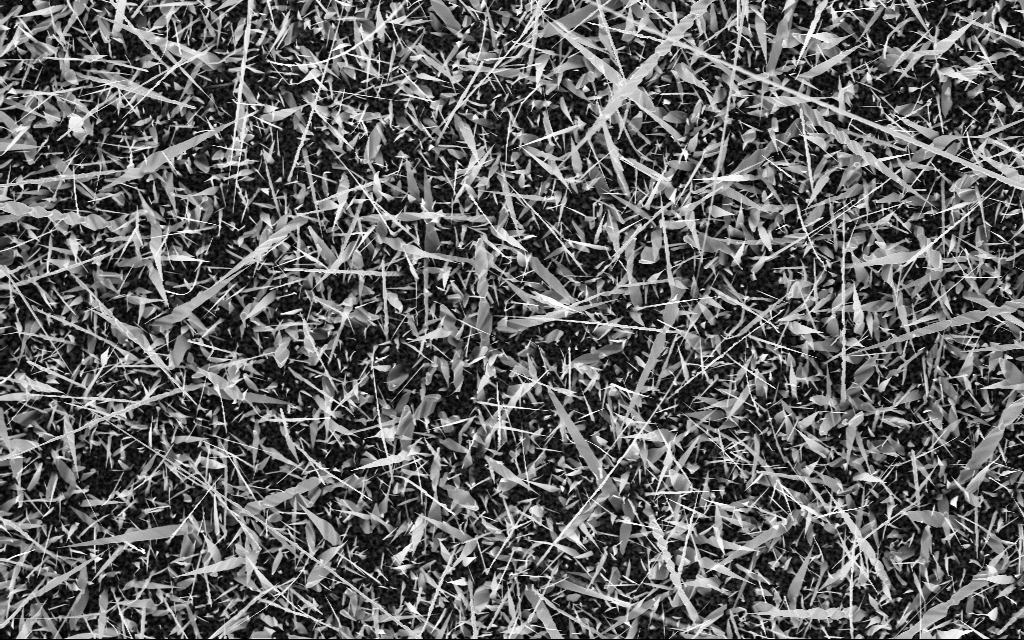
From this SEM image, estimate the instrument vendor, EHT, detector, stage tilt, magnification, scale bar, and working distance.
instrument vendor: Zeiss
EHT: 10 kV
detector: InLens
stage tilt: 0°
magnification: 5 K X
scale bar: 10000 nm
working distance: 6 mm